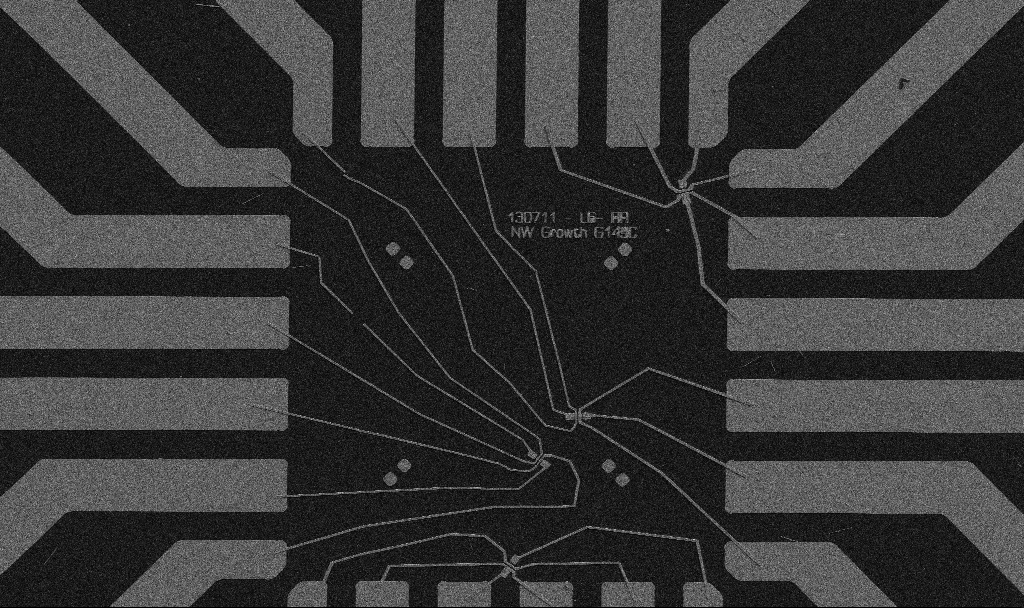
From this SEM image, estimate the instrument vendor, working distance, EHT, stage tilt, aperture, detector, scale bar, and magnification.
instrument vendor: Zeiss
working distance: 10.7 mm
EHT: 5 kV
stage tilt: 0°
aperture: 30 µm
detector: SE2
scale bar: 20000 nm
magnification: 1 K X